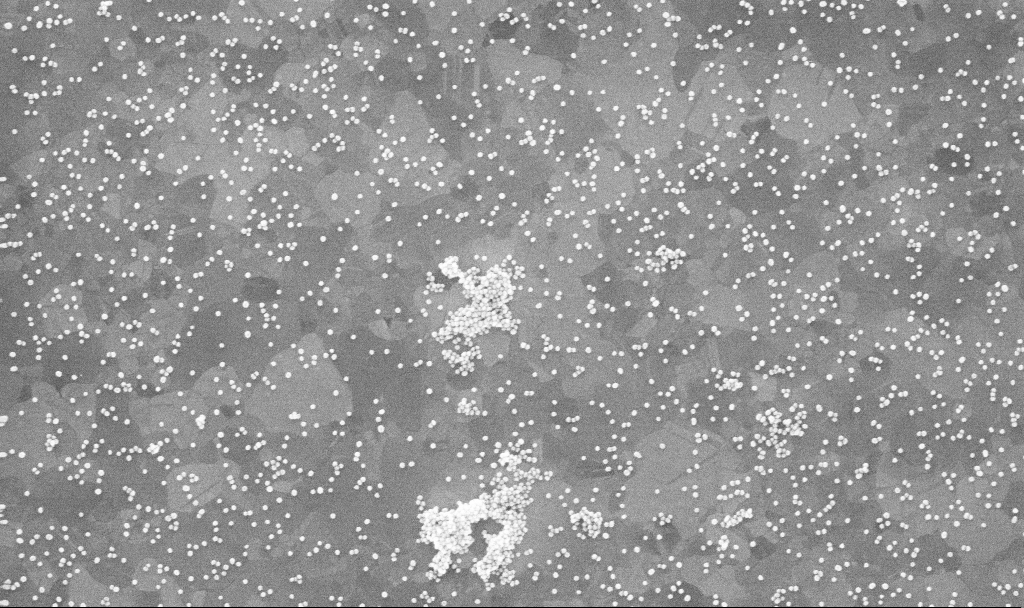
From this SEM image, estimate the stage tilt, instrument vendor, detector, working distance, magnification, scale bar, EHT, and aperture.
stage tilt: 0°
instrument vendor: Zeiss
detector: InLens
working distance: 3.3 mm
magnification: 100 K X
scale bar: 200 nm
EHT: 10 kV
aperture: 30 µm